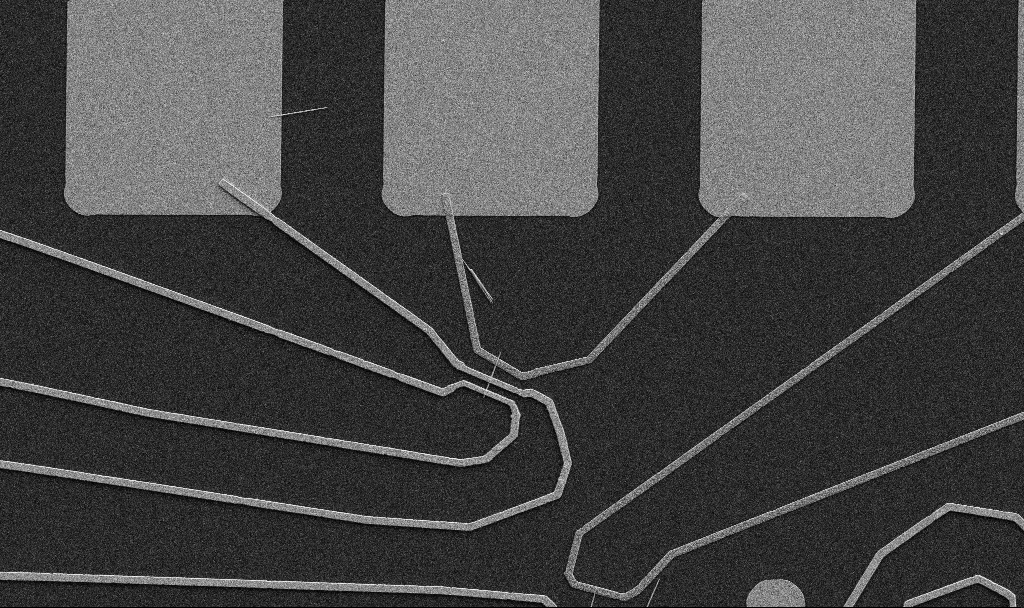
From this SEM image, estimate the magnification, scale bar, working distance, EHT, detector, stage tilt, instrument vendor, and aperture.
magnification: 3.87 K X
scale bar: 10000 nm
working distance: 10.7 mm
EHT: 5 kV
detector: SE2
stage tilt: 0°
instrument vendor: Zeiss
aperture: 30 µm